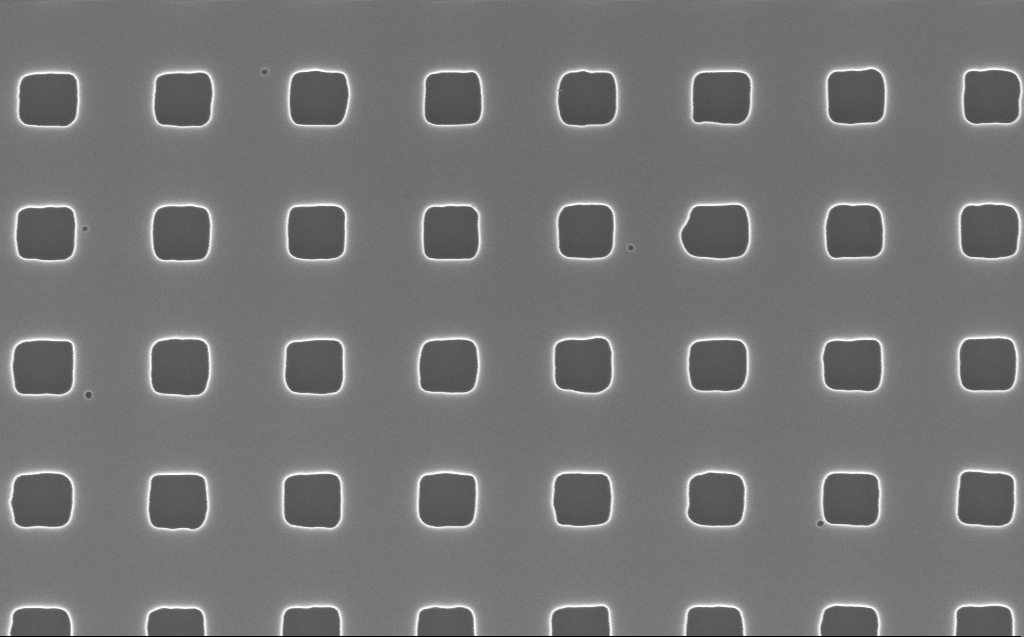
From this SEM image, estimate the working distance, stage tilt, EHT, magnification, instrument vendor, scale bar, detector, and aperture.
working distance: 5 mm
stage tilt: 0°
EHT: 10 kV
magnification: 100 K X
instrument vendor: Zeiss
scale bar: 200 nm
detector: InLens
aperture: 30 µm